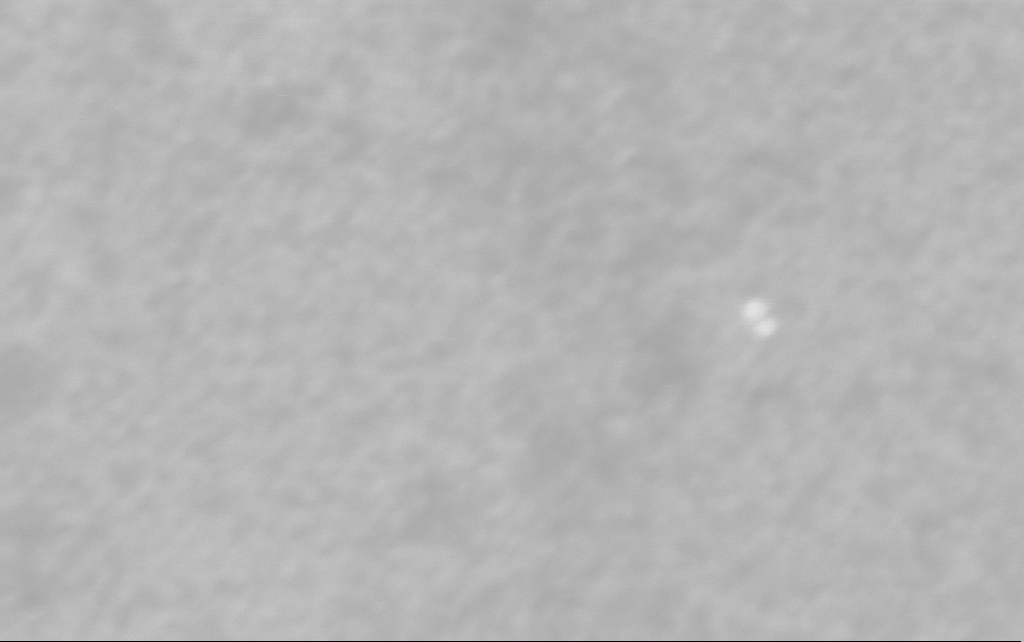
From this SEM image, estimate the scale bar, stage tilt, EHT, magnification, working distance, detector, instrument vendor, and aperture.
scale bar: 100 nm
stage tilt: -0°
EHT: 3 kV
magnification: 499.43 K X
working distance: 2.5 mm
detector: InLens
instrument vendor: Zeiss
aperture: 30 µm